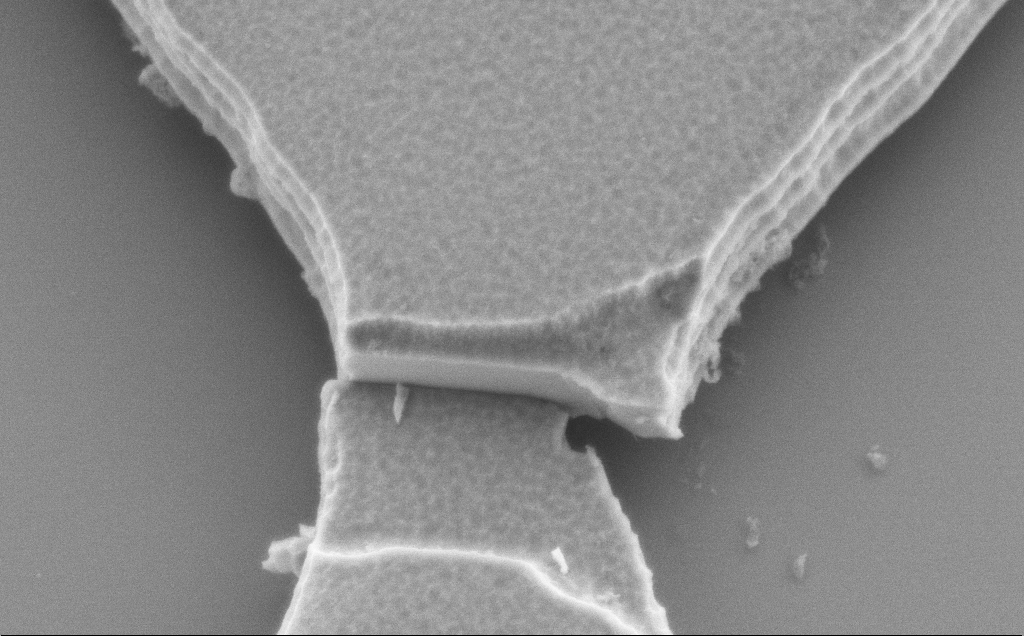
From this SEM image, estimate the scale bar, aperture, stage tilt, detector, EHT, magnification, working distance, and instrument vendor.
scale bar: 1000 nm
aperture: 30 µm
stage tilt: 22.9°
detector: SE2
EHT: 4 kV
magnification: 25.46 K X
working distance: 8 mm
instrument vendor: Zeiss